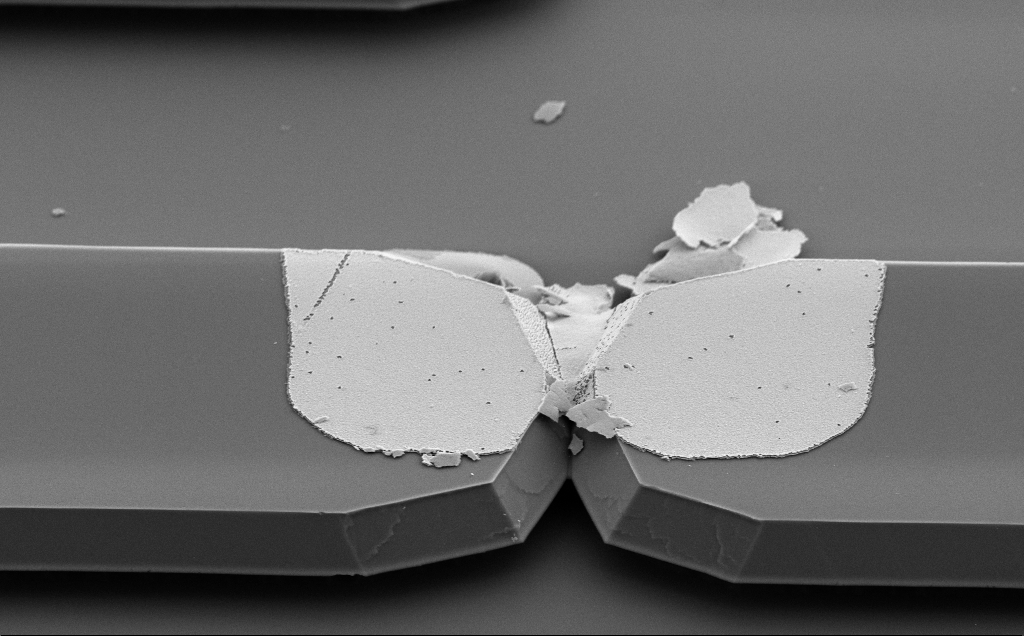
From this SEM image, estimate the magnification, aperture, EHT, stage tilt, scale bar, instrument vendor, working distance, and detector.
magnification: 8.22 K X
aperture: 30 µm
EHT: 5 kV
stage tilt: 50°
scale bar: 2000 nm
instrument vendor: Zeiss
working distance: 10 mm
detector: SE2